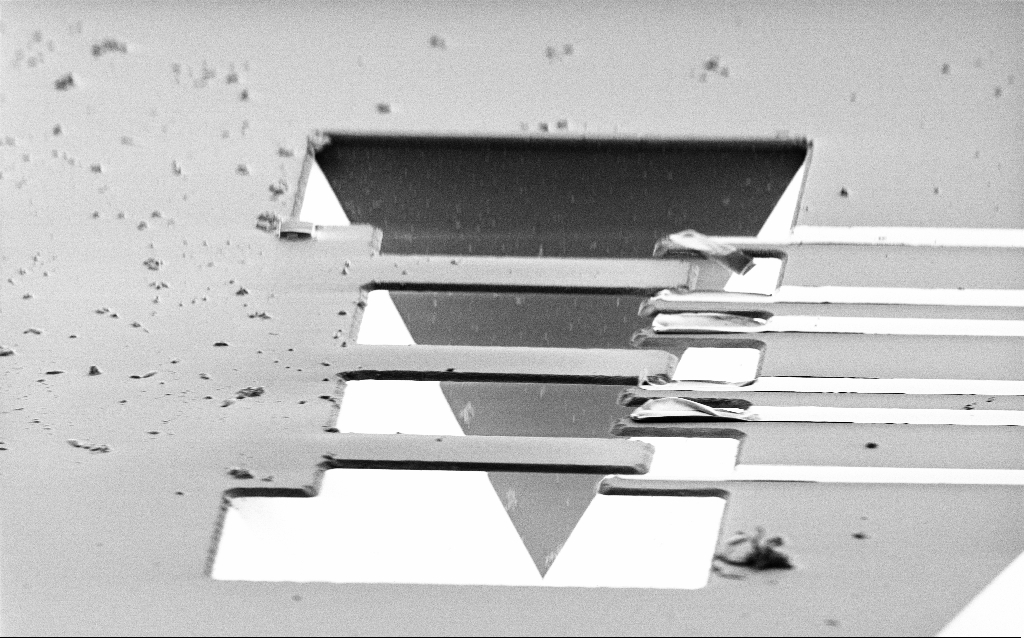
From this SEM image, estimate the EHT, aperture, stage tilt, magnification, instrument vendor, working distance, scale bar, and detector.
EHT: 2 kV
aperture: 30 µm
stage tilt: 70°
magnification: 1.6 K X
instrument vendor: Zeiss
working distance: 9.1 mm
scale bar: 20000 nm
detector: SE2